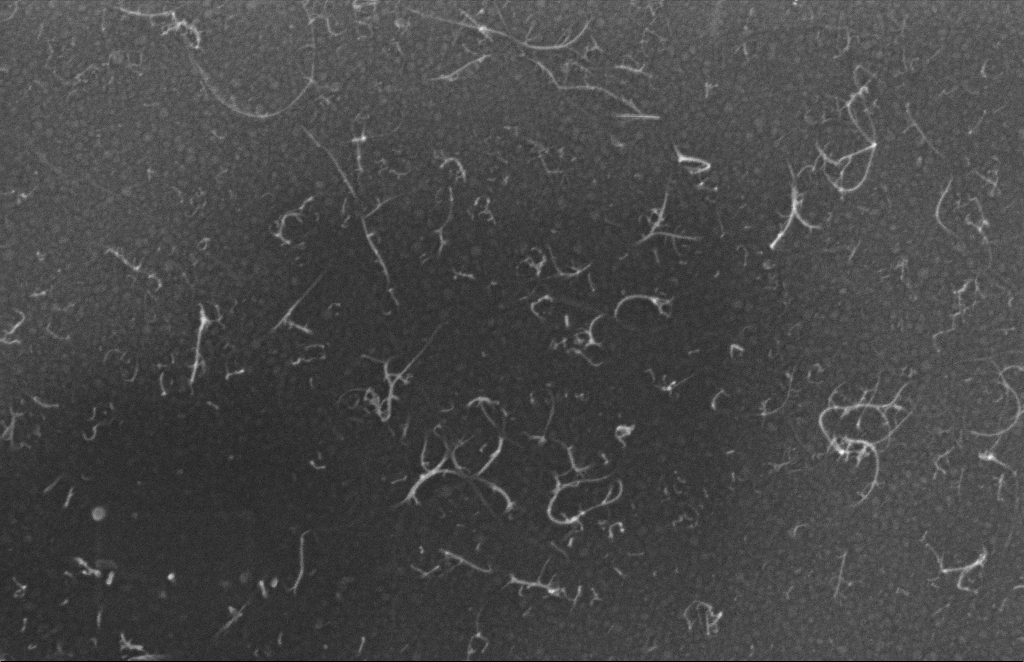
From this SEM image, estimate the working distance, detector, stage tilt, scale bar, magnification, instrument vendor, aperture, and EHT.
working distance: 4 mm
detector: InLens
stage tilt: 0°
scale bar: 100 nm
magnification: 175.33 K X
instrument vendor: Zeiss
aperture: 20 µm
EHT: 5 kV